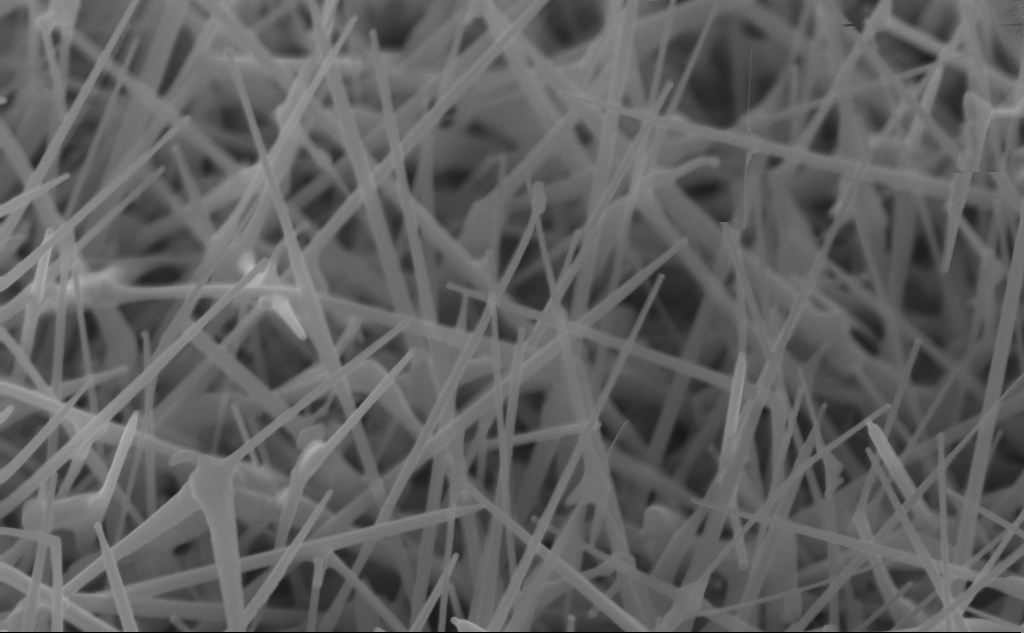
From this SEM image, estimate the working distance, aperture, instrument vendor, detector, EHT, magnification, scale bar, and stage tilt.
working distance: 4 mm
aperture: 30 µm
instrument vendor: Zeiss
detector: InLens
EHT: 10 kV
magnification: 80 K X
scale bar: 200 nm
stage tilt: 45°